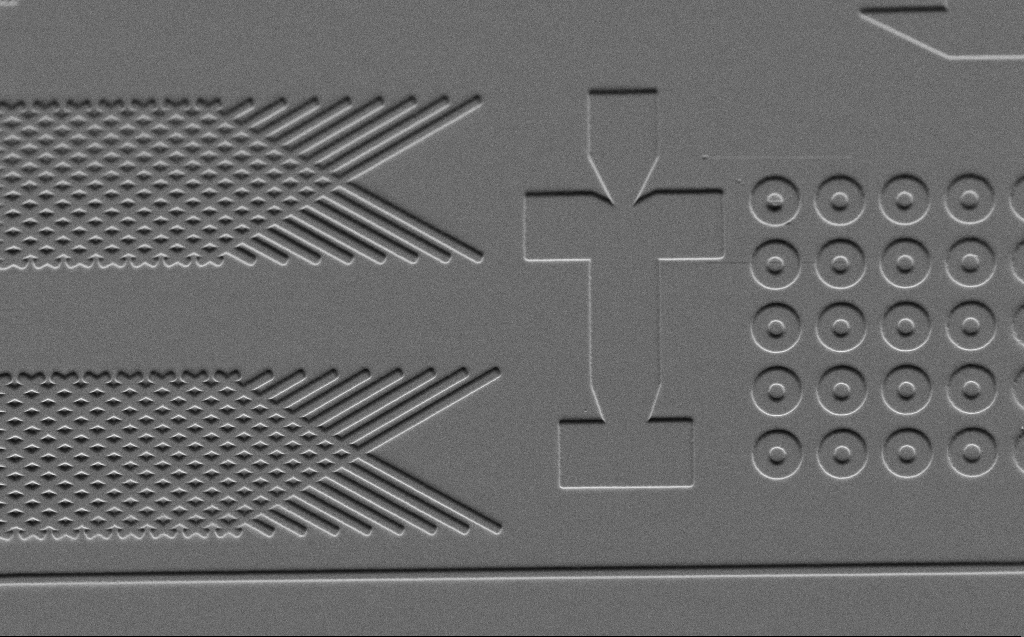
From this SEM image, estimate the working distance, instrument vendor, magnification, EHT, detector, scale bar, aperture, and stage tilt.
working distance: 6 mm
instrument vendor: Zeiss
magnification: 2.39 K X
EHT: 3 kV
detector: SE2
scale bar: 10000 nm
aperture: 30 µm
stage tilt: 45°